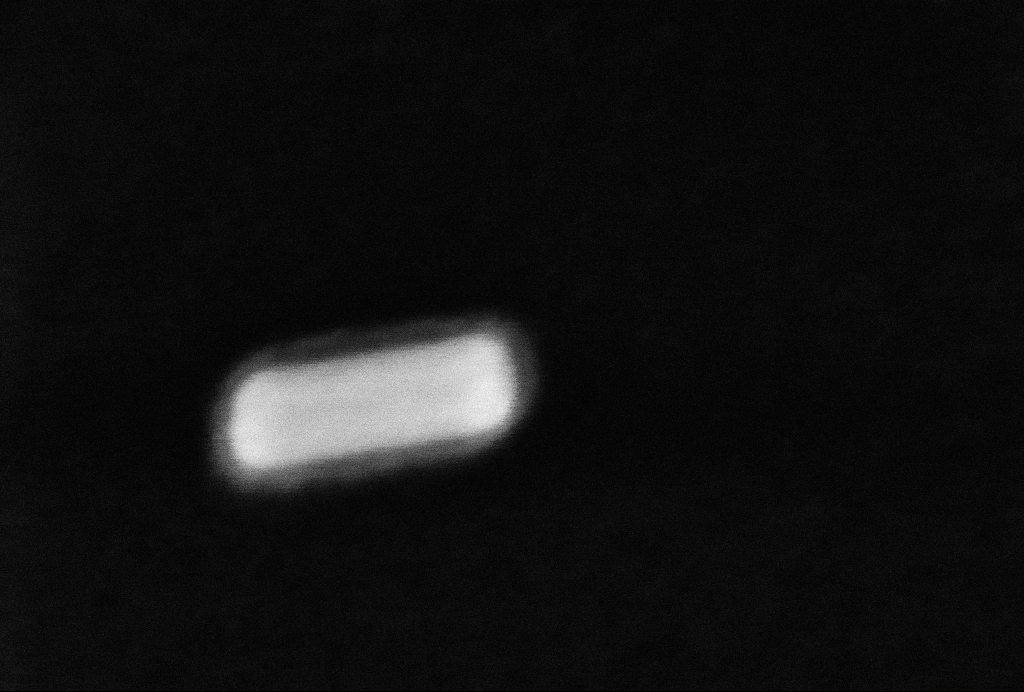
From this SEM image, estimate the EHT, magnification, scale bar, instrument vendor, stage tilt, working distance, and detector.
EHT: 10 kV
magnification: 1492.85 K X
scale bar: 20 nm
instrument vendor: Zeiss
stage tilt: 0°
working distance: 3.2 mm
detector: InLens